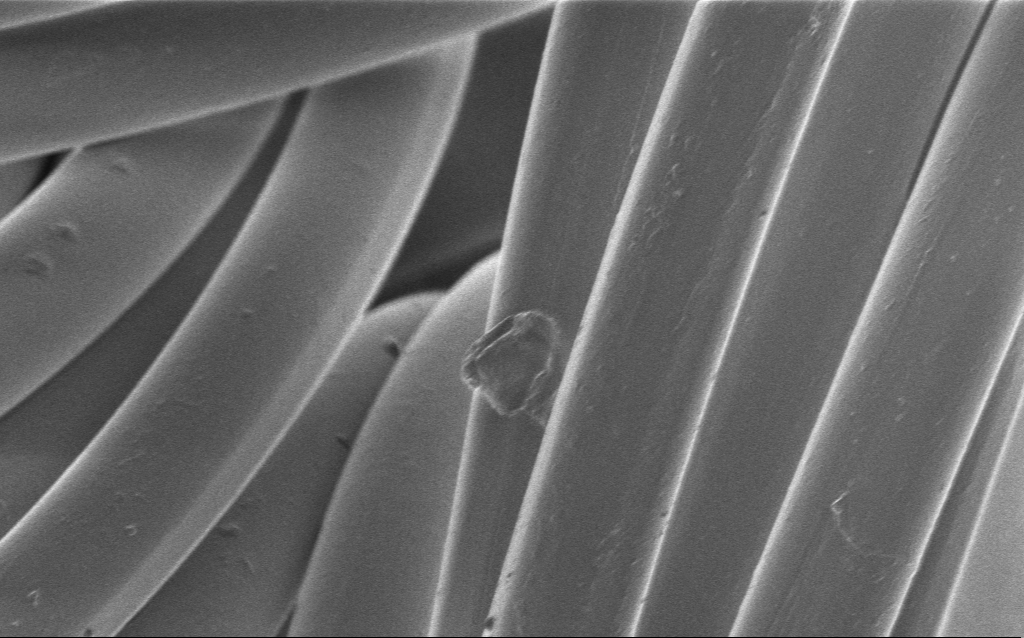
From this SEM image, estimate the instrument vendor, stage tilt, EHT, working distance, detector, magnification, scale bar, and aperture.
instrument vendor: Zeiss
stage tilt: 0°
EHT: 1 kV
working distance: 4 mm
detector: InLens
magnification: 2.33 K X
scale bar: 20000 nm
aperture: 30 µm